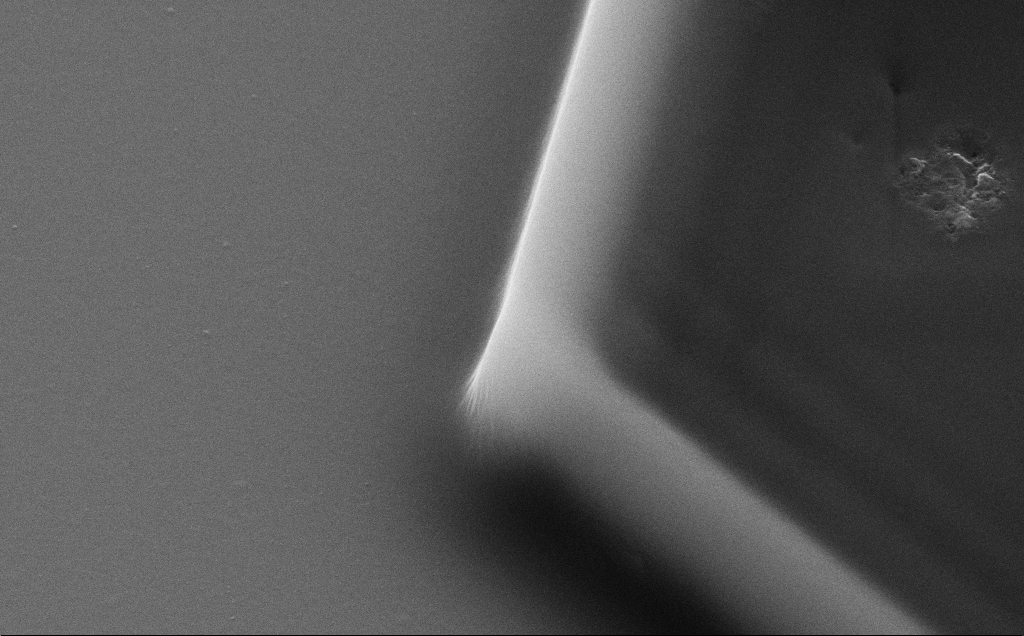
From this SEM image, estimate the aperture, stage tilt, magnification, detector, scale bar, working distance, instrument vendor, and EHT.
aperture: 30 µm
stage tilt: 35°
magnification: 44.7 K X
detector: SE2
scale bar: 1000 nm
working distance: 8 mm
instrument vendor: Zeiss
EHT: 10 kV